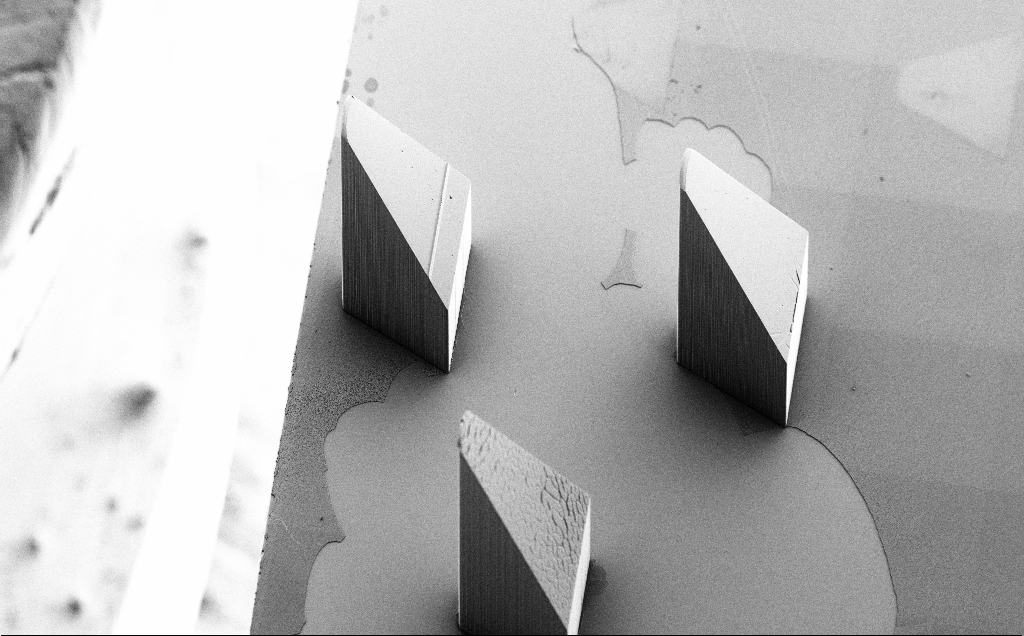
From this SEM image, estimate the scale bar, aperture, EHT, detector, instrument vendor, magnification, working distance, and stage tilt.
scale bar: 100000 nm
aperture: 30 µm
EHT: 5 kV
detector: SE2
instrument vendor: Zeiss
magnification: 0.127 K X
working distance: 9 mm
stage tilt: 40°